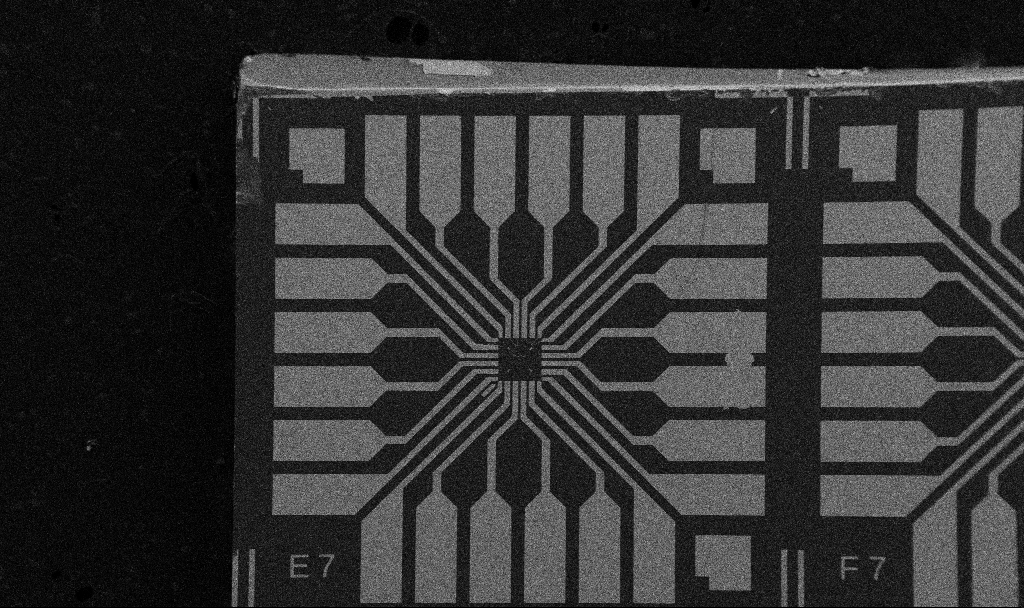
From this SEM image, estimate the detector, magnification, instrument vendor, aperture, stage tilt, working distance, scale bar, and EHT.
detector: SE2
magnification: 0.1 K X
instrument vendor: Zeiss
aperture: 30 µm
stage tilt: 0°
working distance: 10.7 mm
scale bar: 200000 nm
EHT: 5 kV